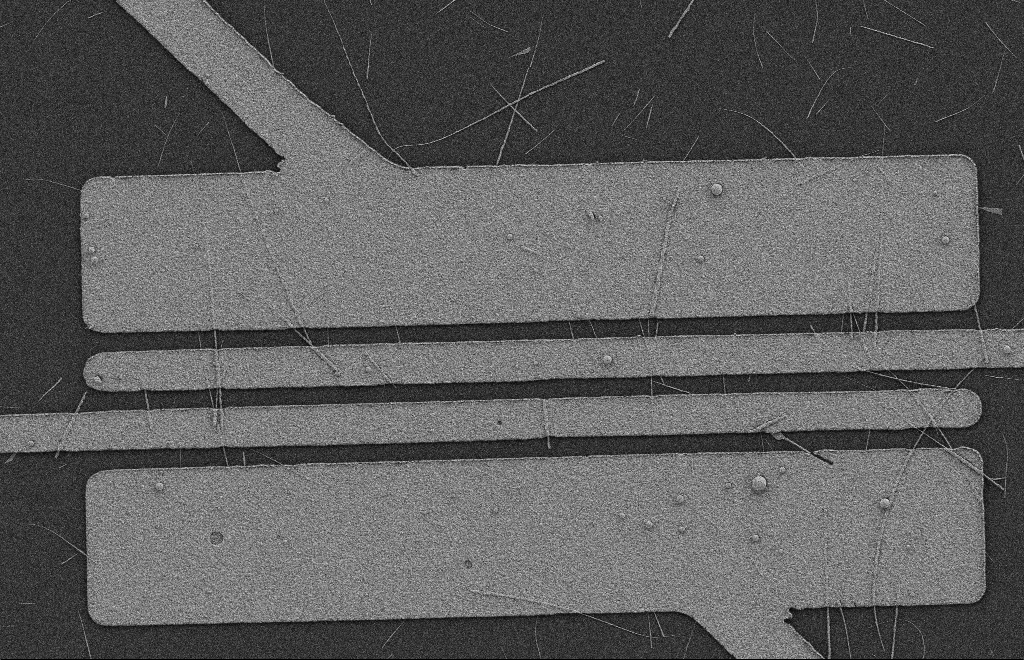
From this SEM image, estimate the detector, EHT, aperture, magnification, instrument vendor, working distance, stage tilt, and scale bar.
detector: SE2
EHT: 2 kV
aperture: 20 µm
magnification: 5.39 K X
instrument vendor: Zeiss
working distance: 8 mm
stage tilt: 0°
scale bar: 2000 nm